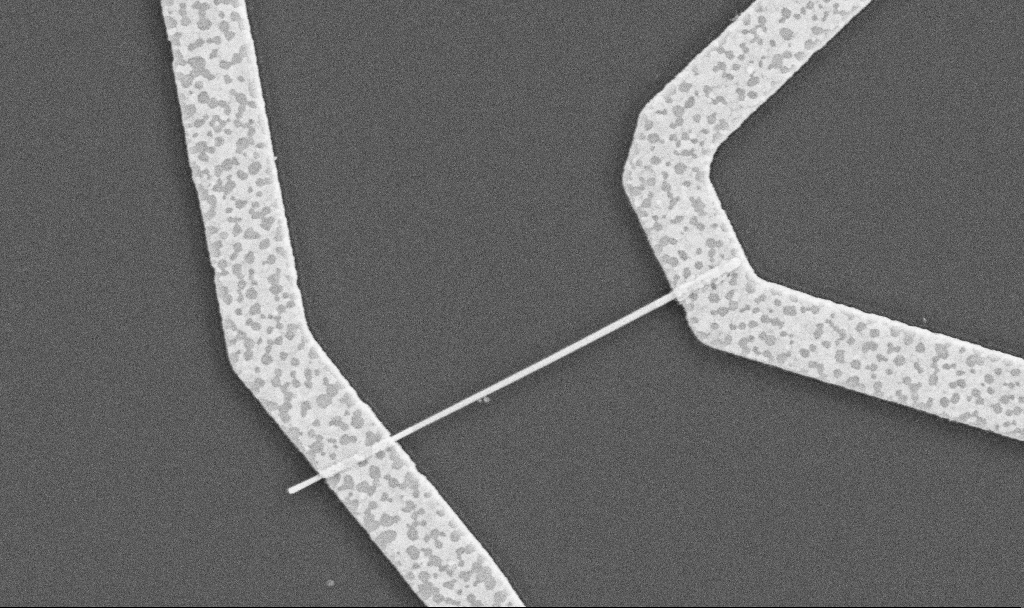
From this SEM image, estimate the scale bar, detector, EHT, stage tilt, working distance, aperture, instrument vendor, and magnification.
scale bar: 1000 nm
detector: SE2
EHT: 5 kV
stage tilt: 0°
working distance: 10.5 mm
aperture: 30 µm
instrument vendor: Zeiss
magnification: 30 K X